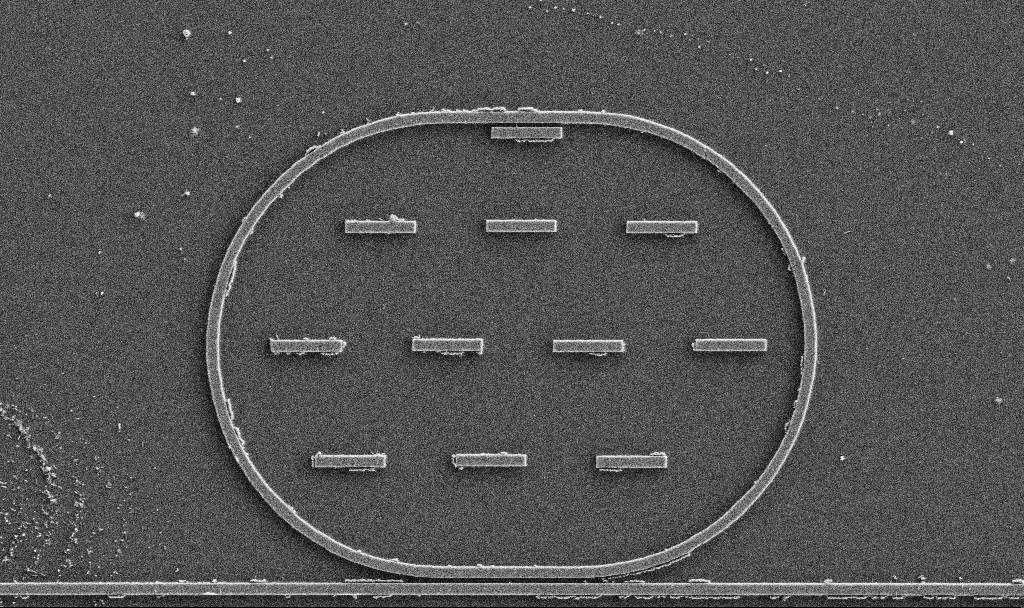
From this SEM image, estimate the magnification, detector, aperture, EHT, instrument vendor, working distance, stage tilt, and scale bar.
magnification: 8.71 K X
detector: SE2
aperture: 30 µm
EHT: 3 kV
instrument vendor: Zeiss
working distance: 3 mm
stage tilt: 0°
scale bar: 2000 nm